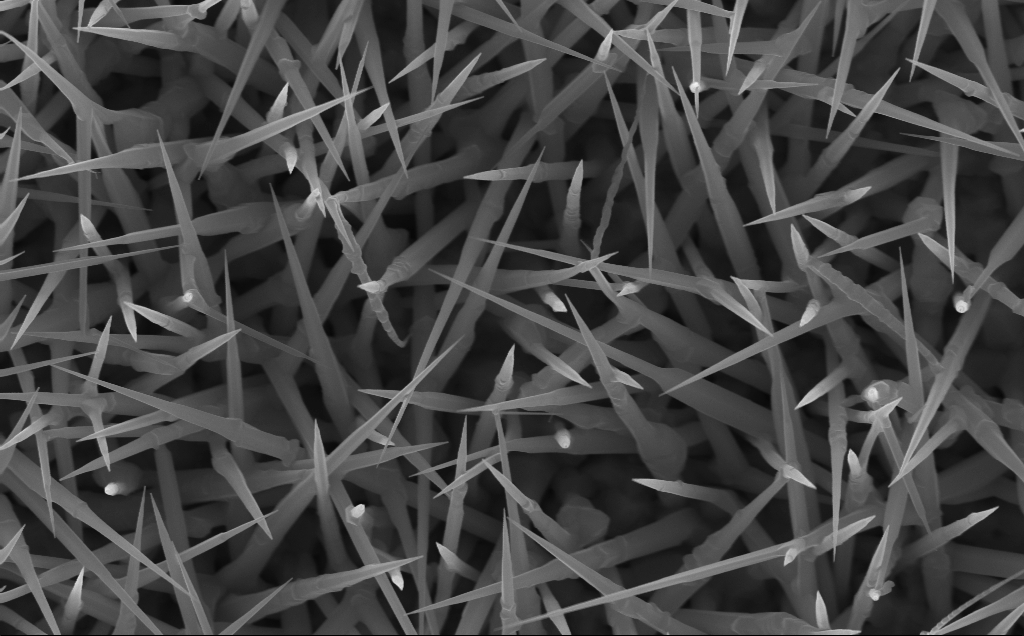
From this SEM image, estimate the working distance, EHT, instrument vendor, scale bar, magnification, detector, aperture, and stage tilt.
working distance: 4 mm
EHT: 10 kV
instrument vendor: Zeiss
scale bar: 1000 nm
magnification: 40 K X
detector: InLens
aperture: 30 µm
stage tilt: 0°